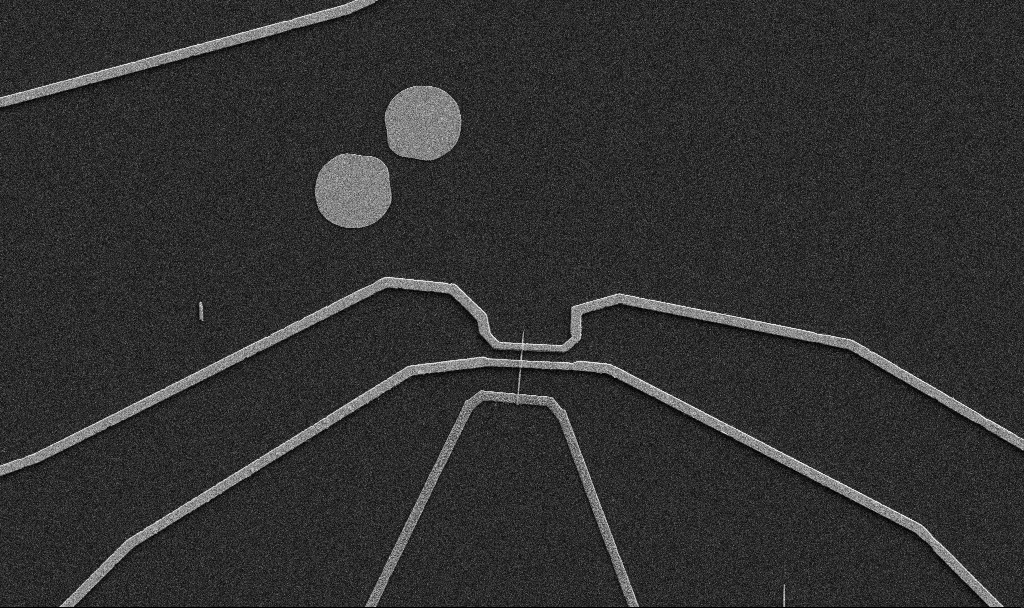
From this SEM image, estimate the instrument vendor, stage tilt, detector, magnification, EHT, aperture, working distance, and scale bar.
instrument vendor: Zeiss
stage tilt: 0°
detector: SE2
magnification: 5 K X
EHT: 5 kV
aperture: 30 µm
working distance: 10.7 mm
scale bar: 10000 nm